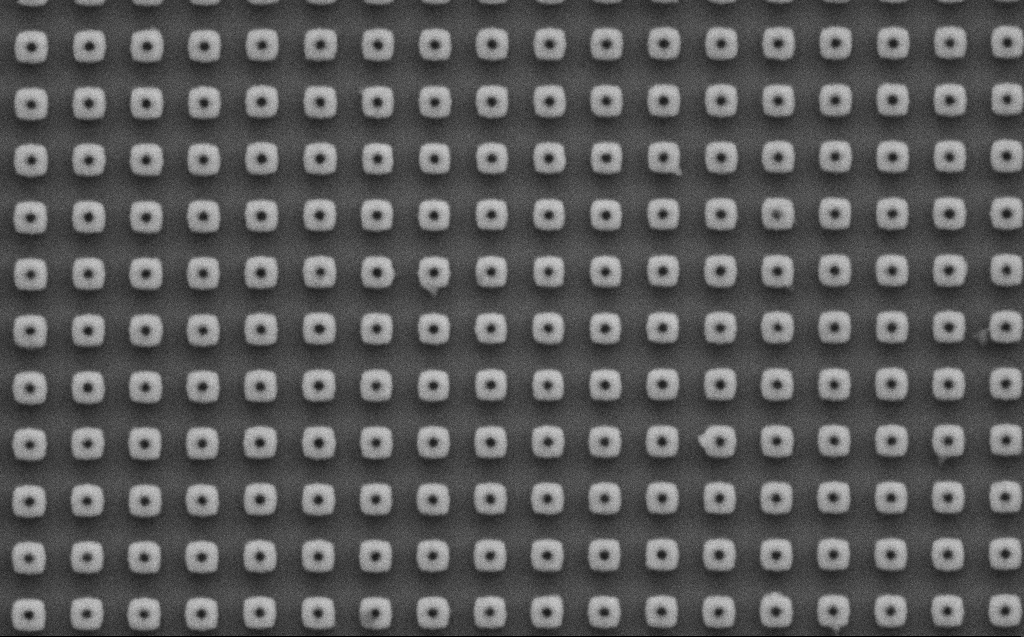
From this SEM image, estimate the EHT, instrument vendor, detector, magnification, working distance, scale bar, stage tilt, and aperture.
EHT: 3 kV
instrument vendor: Zeiss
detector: SE2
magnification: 52.89 K X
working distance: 6 mm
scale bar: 1000 nm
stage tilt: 0°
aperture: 30 µm